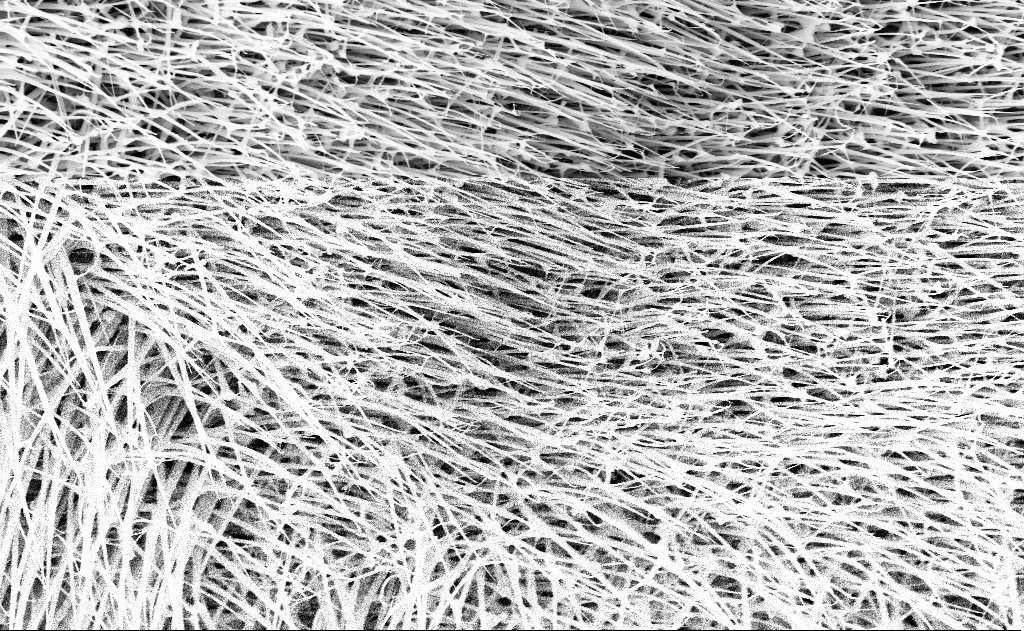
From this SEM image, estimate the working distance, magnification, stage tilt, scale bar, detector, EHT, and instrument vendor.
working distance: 13 mm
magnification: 20 K X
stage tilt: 0°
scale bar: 1000 nm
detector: InLens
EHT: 10 kV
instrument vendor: Zeiss